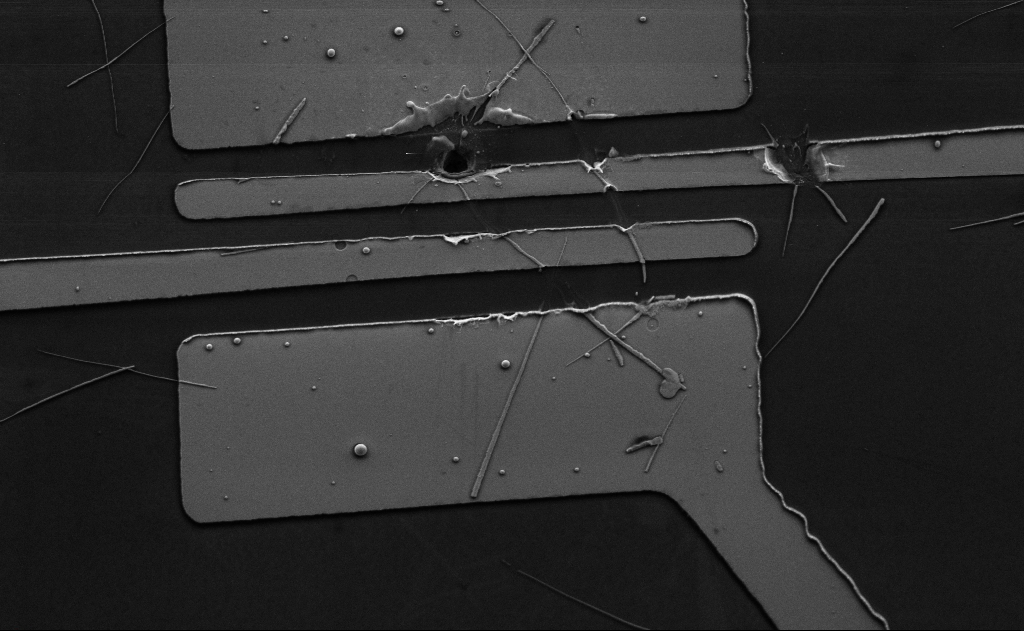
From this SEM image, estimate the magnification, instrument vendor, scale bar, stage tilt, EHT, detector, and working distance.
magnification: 7.26 K X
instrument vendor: Zeiss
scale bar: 2000 nm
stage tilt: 0°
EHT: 5 kV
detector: SE2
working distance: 15 mm